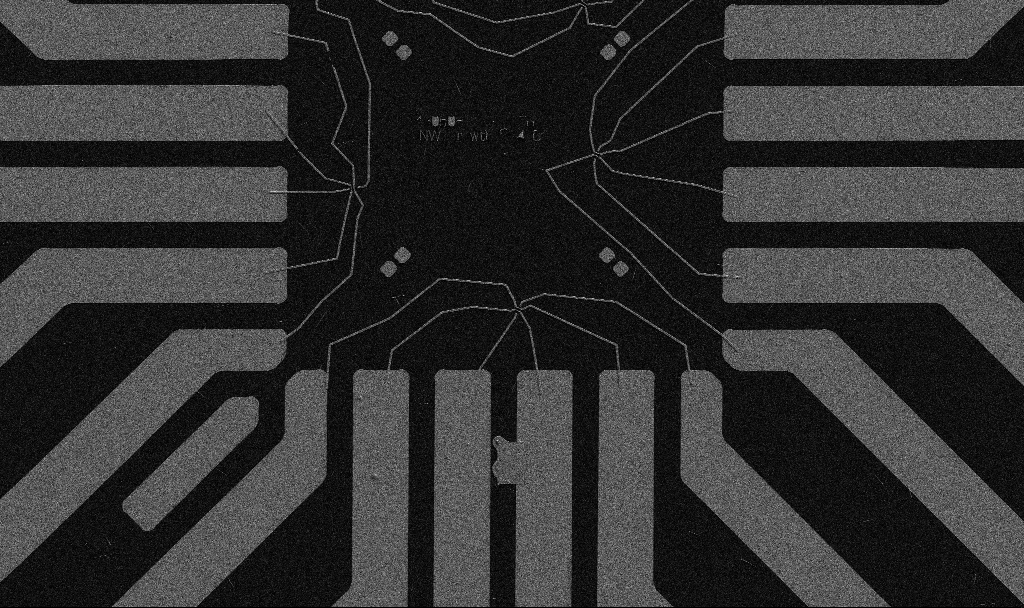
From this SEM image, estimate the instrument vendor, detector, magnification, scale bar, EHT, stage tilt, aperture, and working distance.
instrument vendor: Zeiss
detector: SE2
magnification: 1 K X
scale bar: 20000 nm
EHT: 5 kV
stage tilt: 0°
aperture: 30 µm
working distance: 10.7 mm